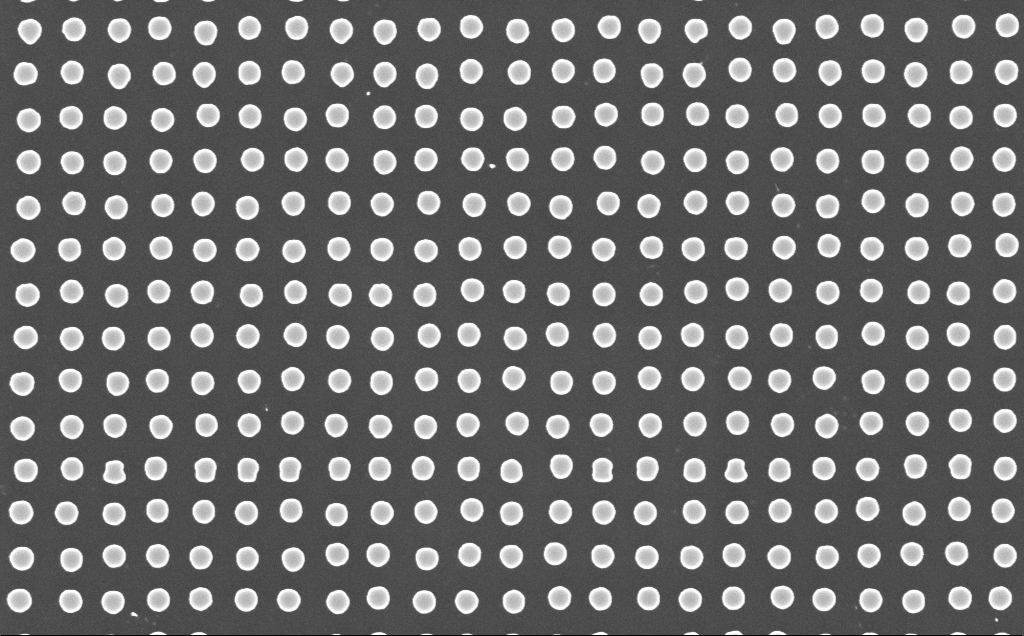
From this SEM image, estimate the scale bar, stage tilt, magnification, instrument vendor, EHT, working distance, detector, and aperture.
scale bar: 1000 nm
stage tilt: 0°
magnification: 40.88 K X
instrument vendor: Zeiss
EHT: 10 kV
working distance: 6 mm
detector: InLens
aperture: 30 µm